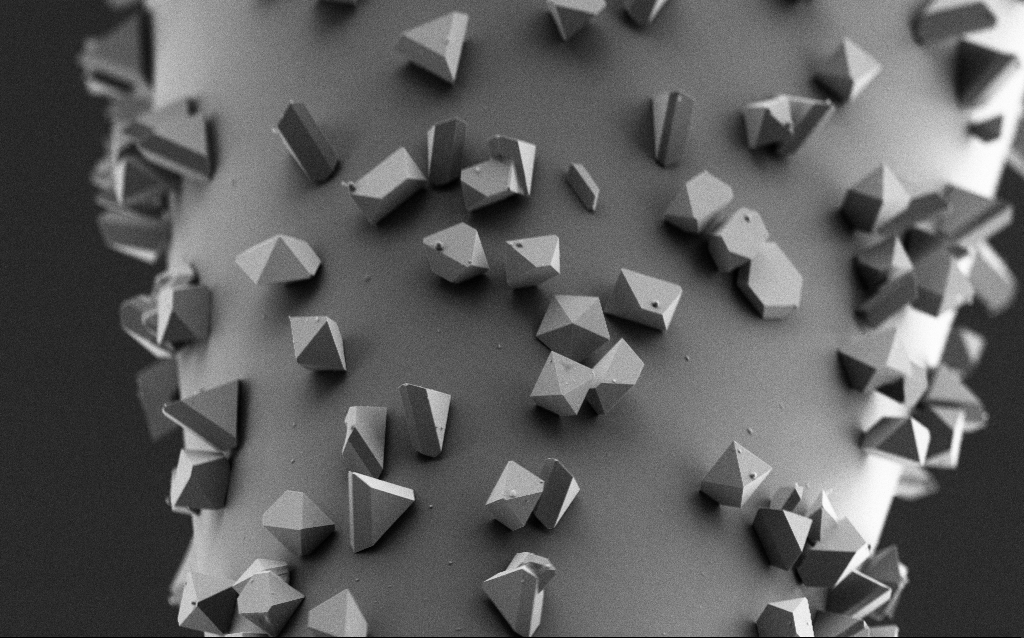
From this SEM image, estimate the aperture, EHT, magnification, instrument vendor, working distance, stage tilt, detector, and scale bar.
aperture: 30 µm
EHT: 2 kV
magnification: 10 K X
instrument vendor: Zeiss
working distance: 4 mm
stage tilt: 45°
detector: SE2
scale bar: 2000 nm